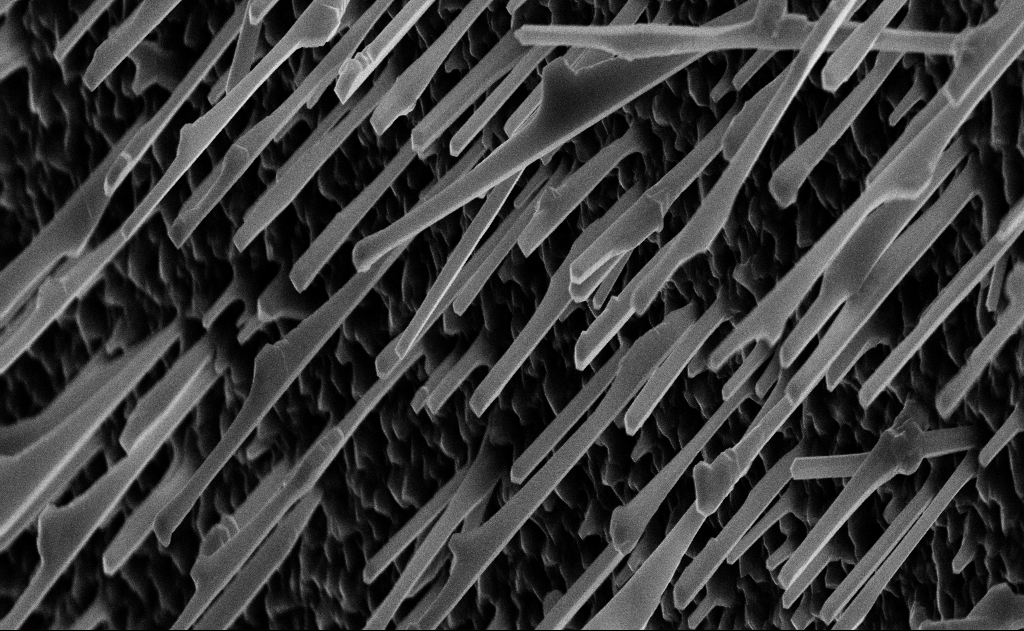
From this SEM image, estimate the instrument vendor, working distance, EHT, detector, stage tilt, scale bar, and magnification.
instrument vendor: Zeiss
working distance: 15 mm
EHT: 10 kV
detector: InLens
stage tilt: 0°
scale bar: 2000 nm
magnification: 20 K X